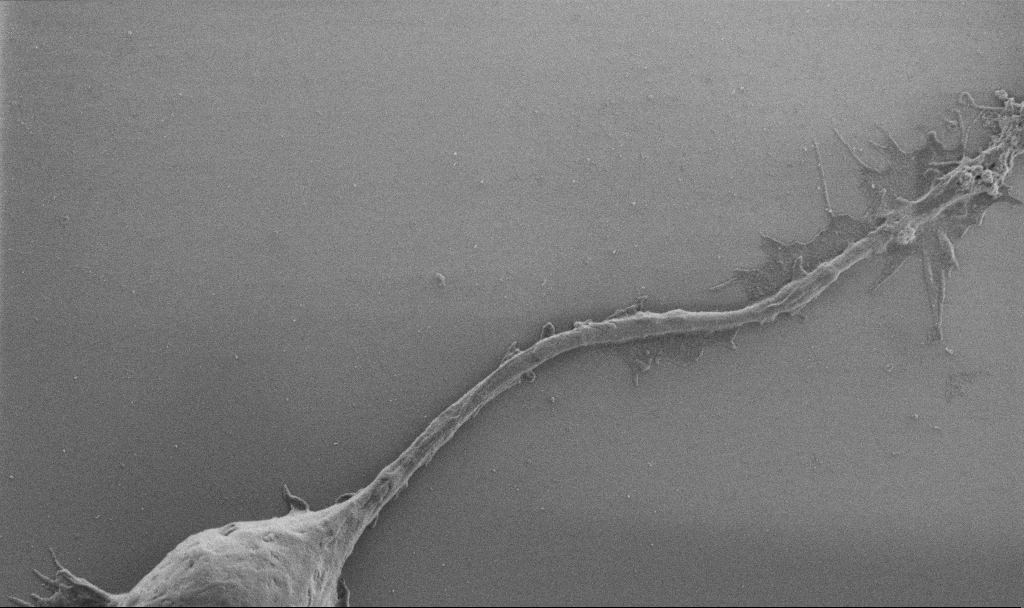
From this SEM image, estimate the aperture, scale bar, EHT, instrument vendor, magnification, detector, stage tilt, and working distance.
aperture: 30 µm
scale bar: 2000 nm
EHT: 1 kV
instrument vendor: Zeiss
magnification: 10 K X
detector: SE2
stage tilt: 0°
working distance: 6.9 mm